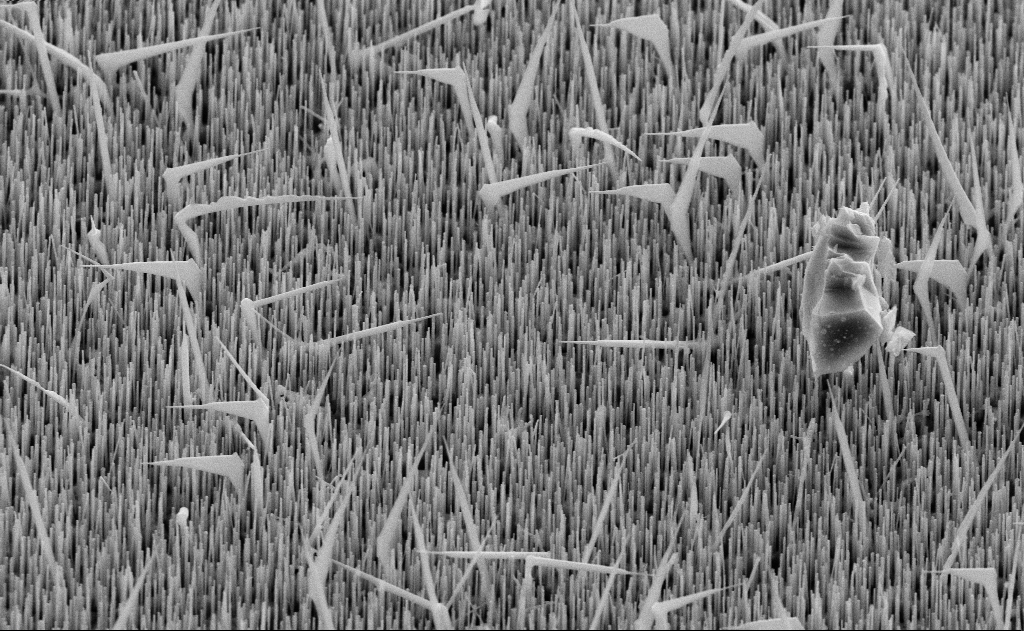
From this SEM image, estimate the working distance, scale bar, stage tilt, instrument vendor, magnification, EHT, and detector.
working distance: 11 mm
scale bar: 1000 nm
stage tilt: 45°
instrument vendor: Zeiss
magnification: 20 K X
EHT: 10 kV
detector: SE2